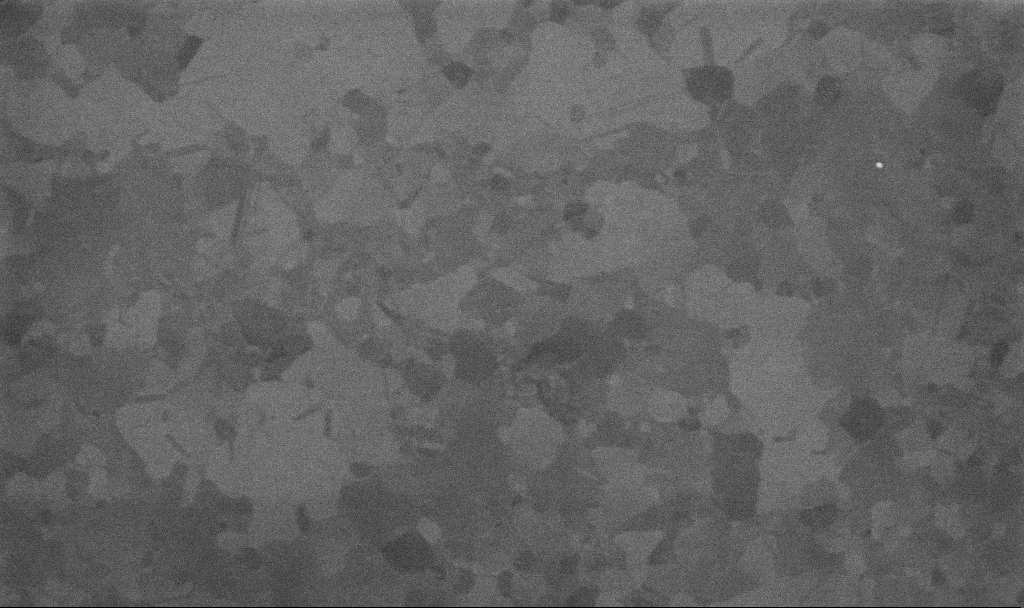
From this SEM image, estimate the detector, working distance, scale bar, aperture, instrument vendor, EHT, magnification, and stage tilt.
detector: InLens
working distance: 3.3 mm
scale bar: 200 nm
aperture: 30 µm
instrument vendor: Zeiss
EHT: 10 kV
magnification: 100 K X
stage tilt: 0°